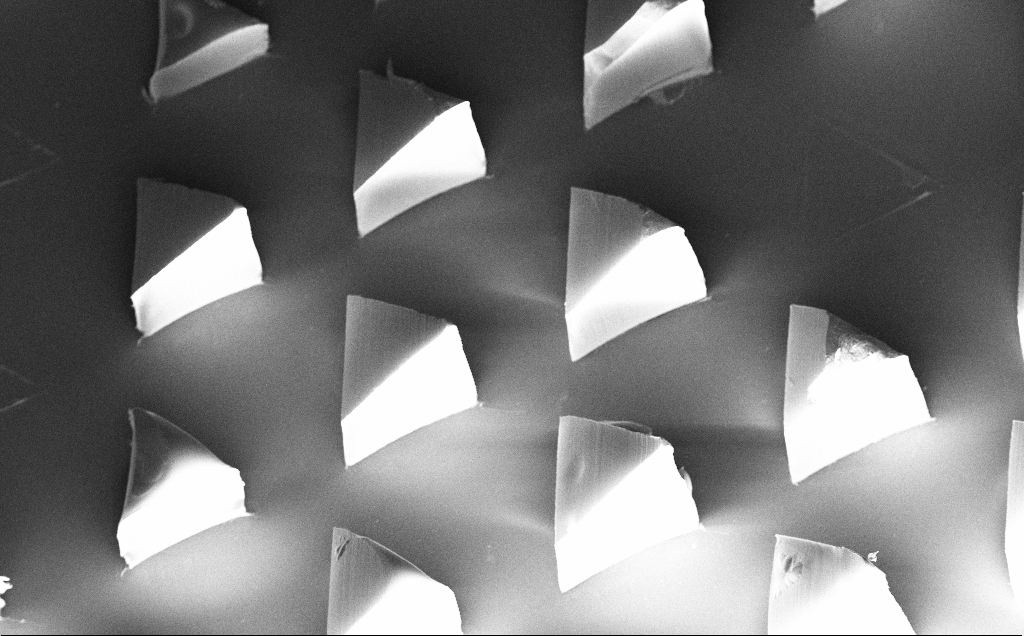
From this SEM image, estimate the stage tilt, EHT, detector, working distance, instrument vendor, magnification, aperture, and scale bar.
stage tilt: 20°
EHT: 10 kV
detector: InLens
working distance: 10 mm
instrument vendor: Zeiss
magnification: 0.212 K X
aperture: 30 µm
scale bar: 200000 nm